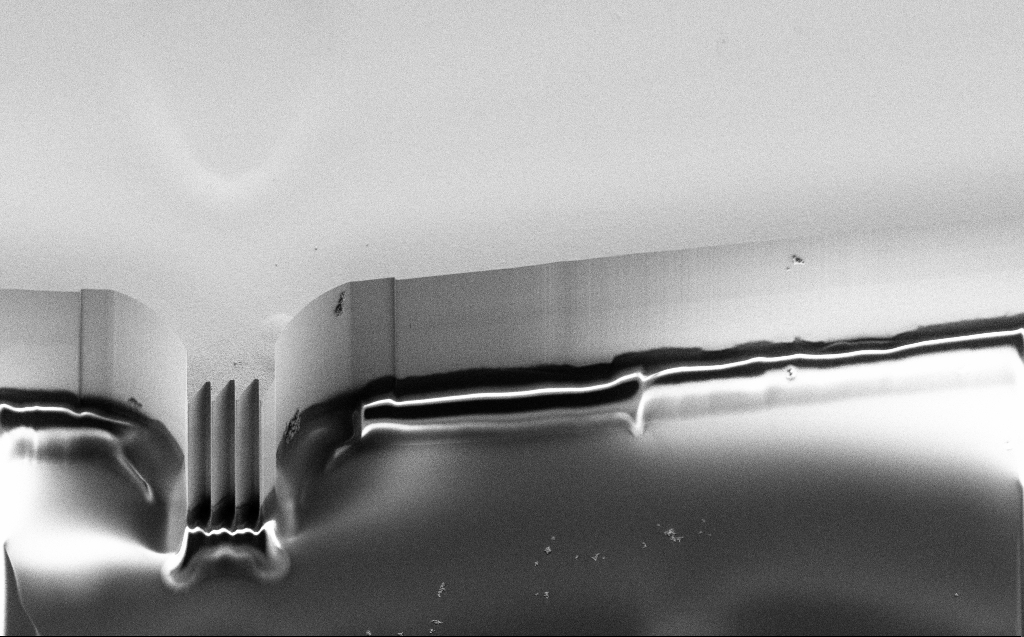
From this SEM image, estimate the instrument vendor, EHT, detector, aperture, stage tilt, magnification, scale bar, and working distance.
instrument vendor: Zeiss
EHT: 5 kV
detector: SE2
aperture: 30 µm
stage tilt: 45°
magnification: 0.786 K X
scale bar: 20000 nm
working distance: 5 mm